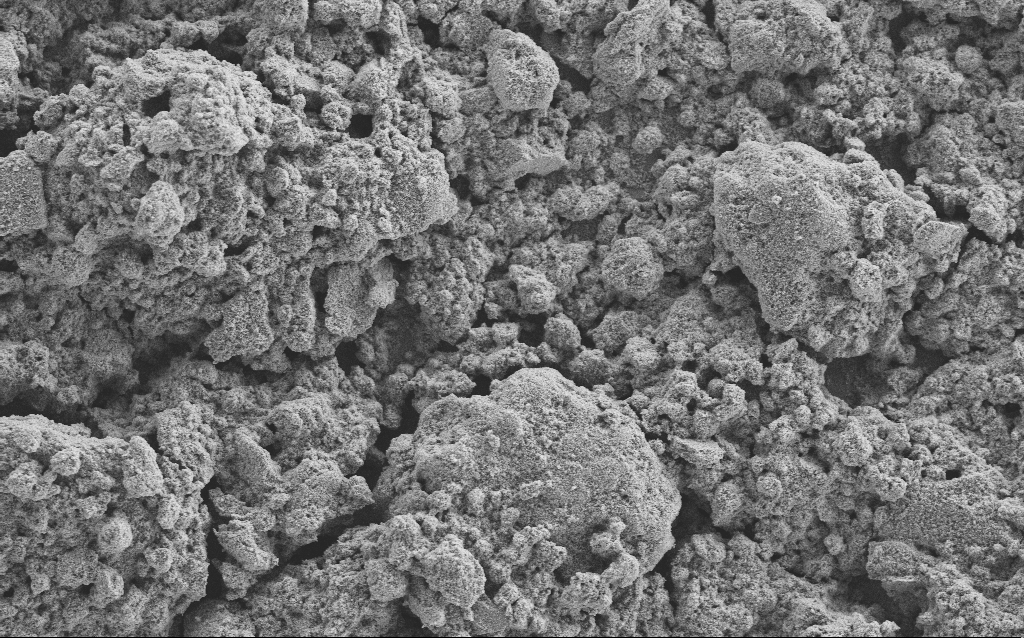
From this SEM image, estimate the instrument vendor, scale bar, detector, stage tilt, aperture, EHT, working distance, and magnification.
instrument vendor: Zeiss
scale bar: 10000 nm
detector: SE2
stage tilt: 0°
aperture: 30 µm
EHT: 5 kV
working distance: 4.1 mm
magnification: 1.23 K X